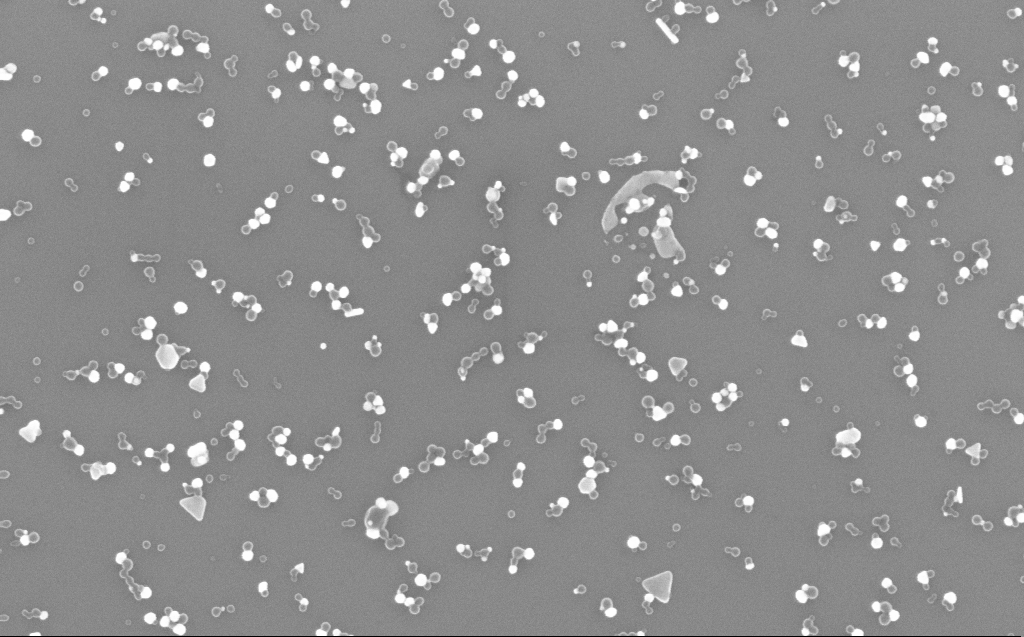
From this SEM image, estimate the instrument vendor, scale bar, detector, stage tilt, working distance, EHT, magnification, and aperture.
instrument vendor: Zeiss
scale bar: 200 nm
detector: InLens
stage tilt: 0°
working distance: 3 mm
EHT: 10 kV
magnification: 80 K X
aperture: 30 µm